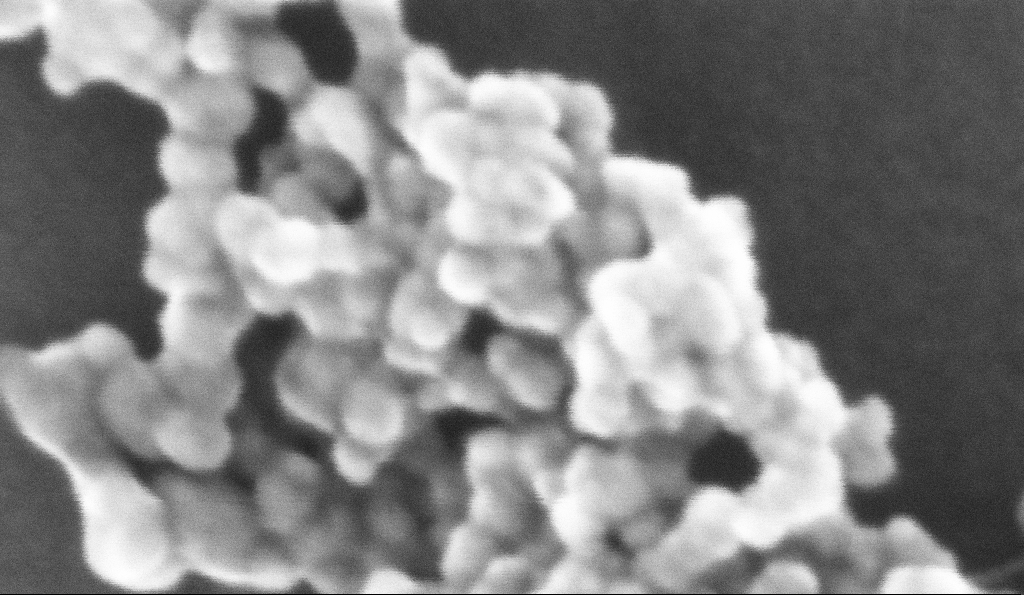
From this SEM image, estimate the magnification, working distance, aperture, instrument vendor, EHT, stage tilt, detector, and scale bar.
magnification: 1244.24 K X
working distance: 5.2 mm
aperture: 30 µm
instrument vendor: Zeiss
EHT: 10 kV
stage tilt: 0°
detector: InLens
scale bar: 20 nm